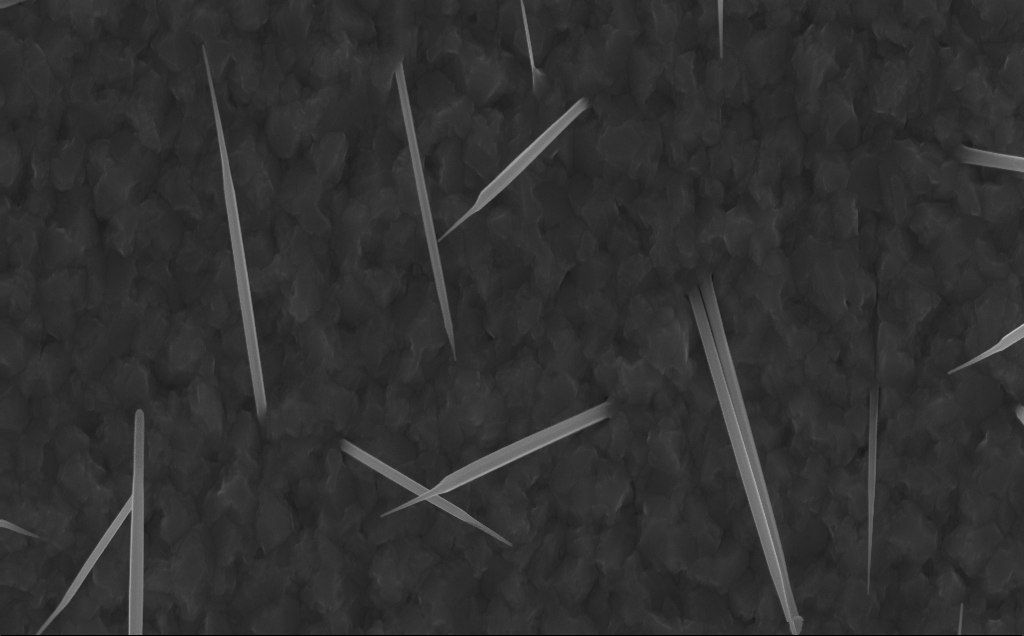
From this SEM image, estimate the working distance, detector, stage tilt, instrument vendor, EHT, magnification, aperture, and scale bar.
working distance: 5 mm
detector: InLens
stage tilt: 0°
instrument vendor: Zeiss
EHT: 10 kV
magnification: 40 K X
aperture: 30 µm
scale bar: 1000 nm